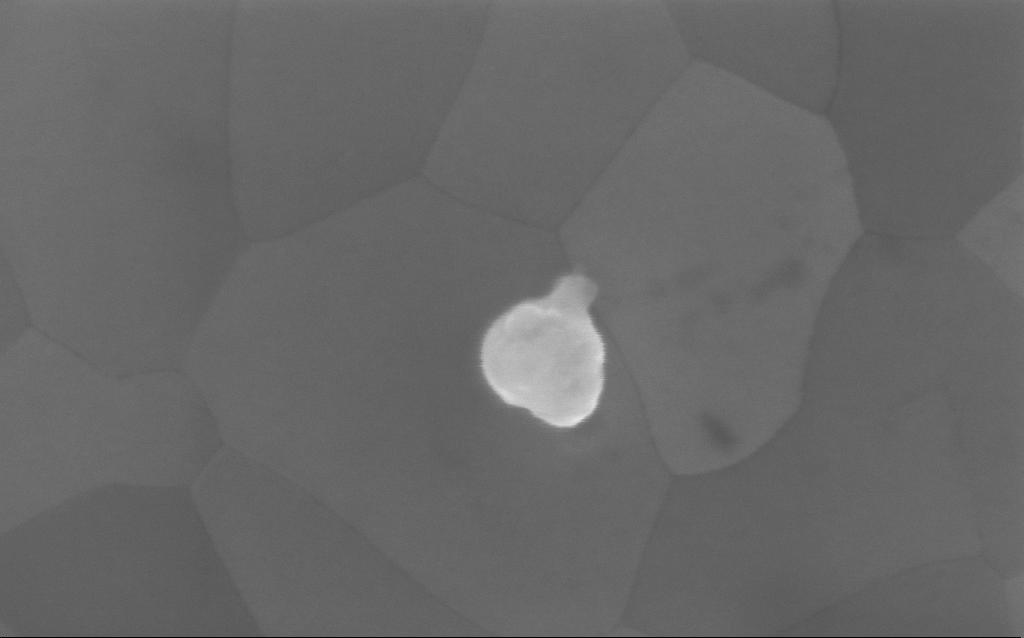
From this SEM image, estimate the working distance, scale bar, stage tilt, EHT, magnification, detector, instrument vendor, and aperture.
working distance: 4 mm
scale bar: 100 nm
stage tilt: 0°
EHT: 5 kV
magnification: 267.42 K X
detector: InLens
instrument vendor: Zeiss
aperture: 30 µm